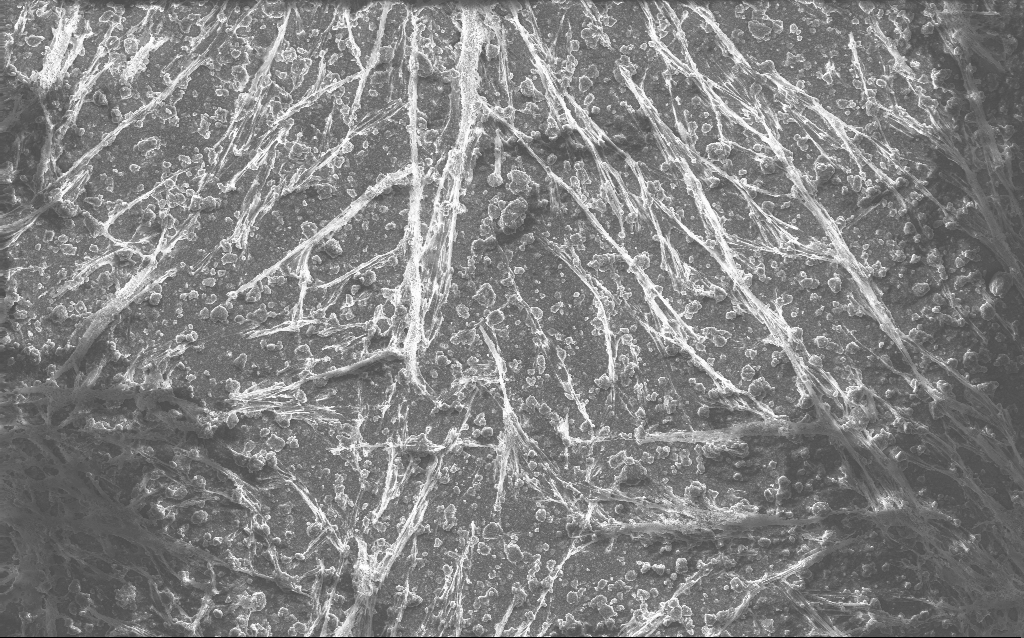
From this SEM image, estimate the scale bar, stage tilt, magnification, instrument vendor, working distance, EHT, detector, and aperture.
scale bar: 20000 nm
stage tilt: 0°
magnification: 0.792 K X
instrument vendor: Zeiss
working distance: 2.8 mm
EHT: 10 kV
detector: InLens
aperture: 30 µm